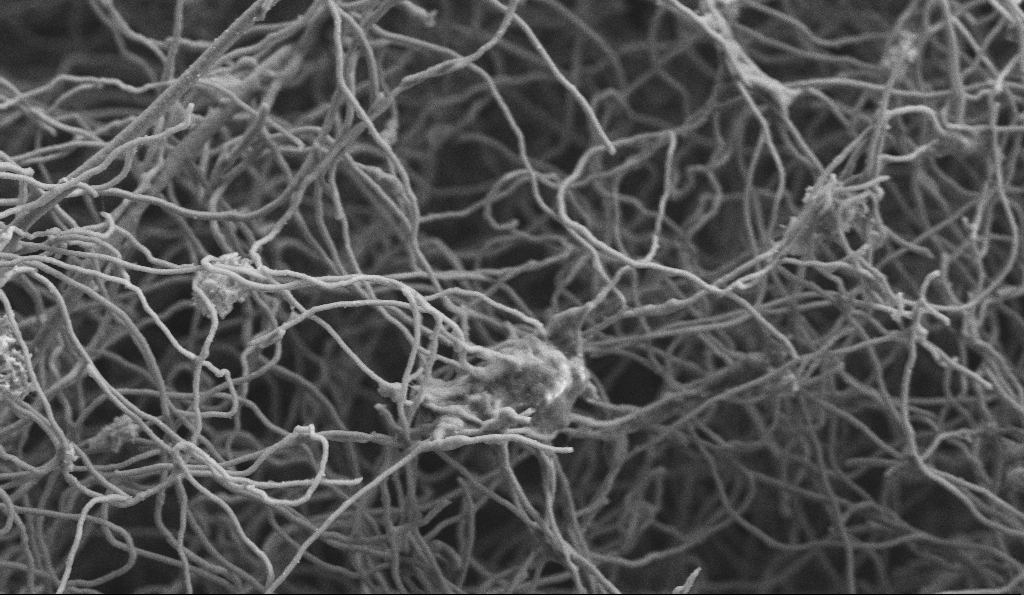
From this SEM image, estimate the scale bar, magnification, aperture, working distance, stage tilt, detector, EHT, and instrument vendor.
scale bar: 1000 nm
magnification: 15 K X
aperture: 30 µm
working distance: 5.1 mm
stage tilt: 0°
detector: SE2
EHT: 3 kV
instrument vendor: Zeiss